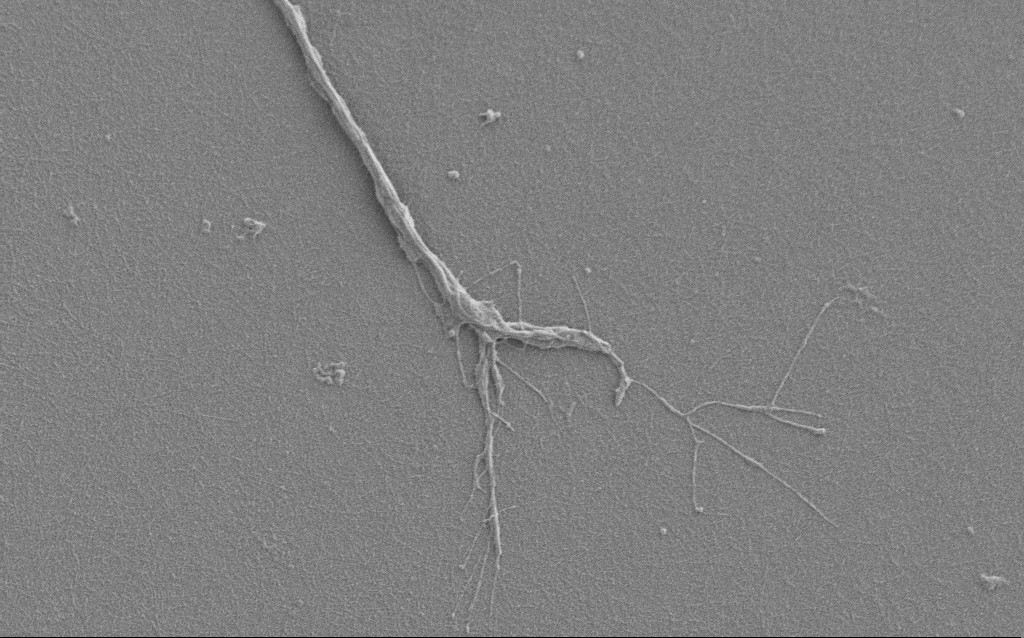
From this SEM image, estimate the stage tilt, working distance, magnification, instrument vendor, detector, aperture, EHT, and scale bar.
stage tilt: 0°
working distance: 6 mm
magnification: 6 K X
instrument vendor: Zeiss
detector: SE2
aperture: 30 µm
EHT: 1 kV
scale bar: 10000 nm